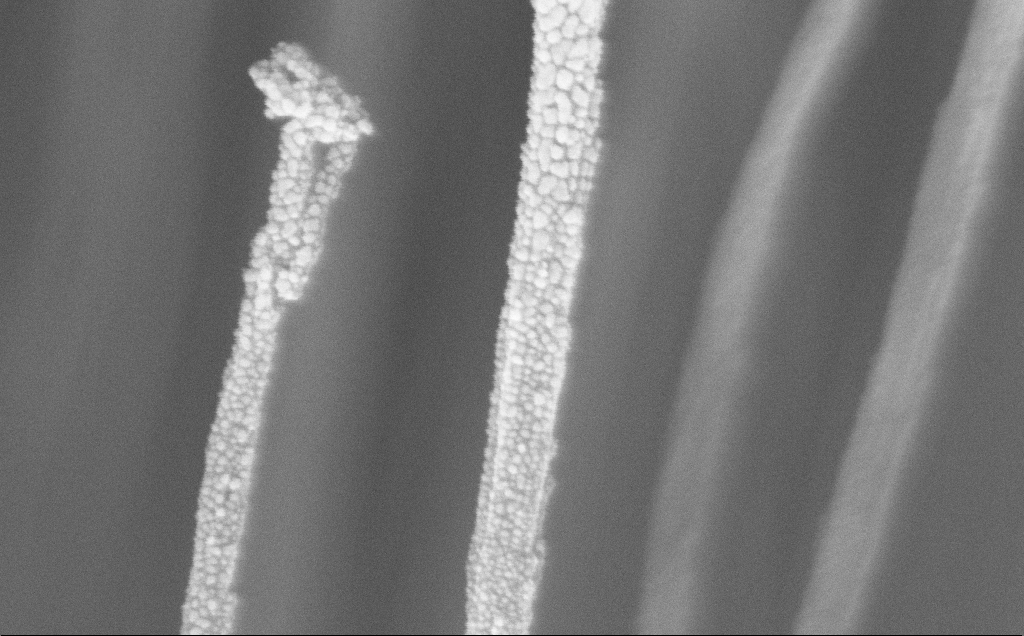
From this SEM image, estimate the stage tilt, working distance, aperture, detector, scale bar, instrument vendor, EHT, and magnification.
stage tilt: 0°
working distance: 11 mm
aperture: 30 µm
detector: InLens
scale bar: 200 nm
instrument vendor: Zeiss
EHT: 5 kV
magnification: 163.96 K X